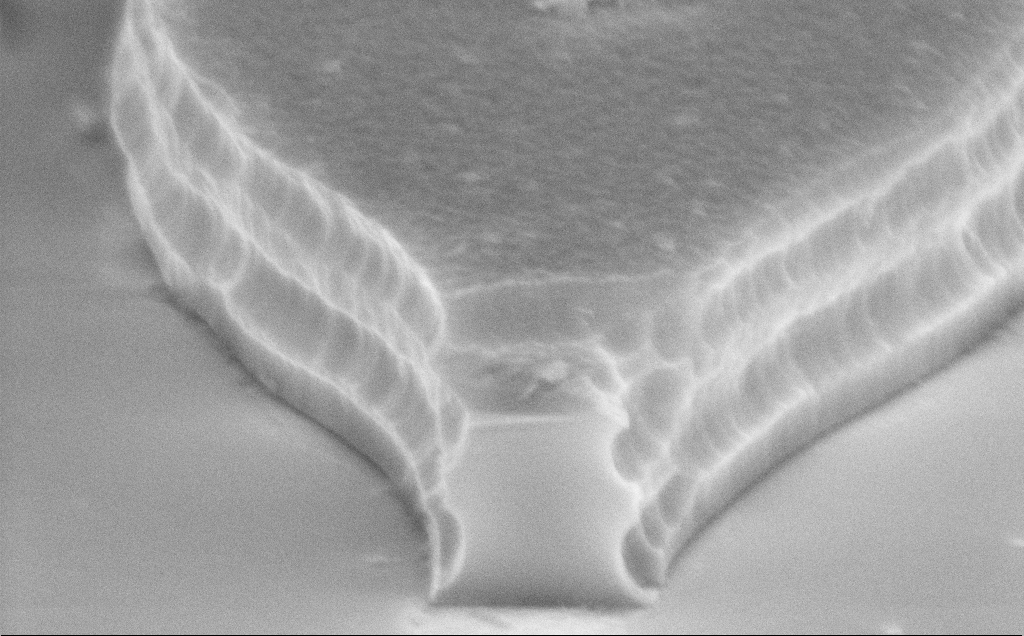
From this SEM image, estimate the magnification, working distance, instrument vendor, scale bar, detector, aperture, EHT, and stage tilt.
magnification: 44.58 K X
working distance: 12 mm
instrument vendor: Zeiss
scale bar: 1000 nm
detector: InLens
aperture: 30 µm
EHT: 8 kV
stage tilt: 70°